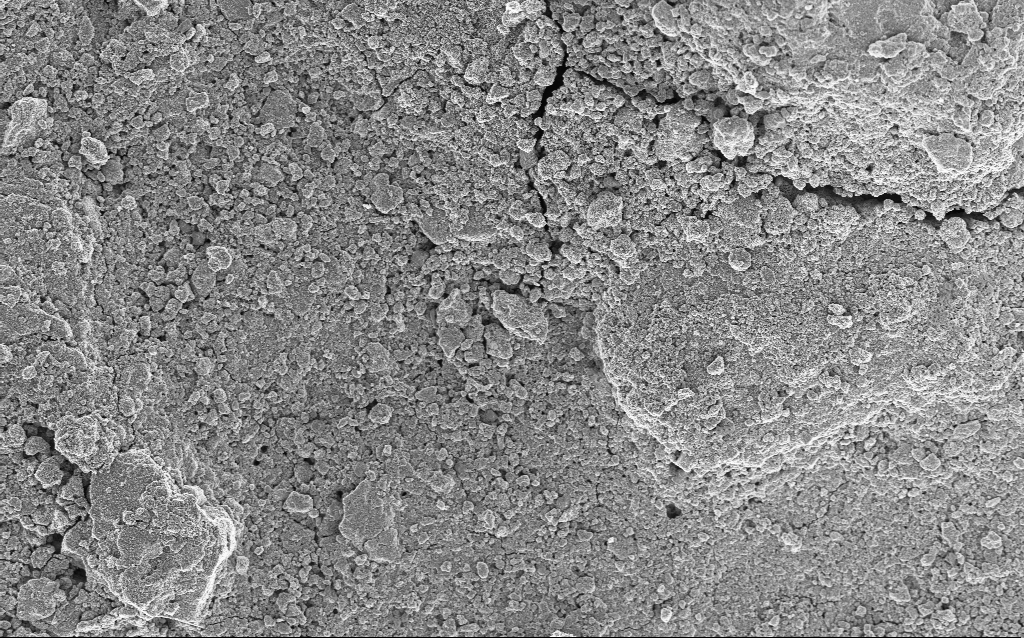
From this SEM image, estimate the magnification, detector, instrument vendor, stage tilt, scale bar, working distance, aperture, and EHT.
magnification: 1.23 K X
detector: InLens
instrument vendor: Zeiss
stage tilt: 0°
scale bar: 10000 nm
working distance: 4.5 mm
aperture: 30 µm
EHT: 5 kV